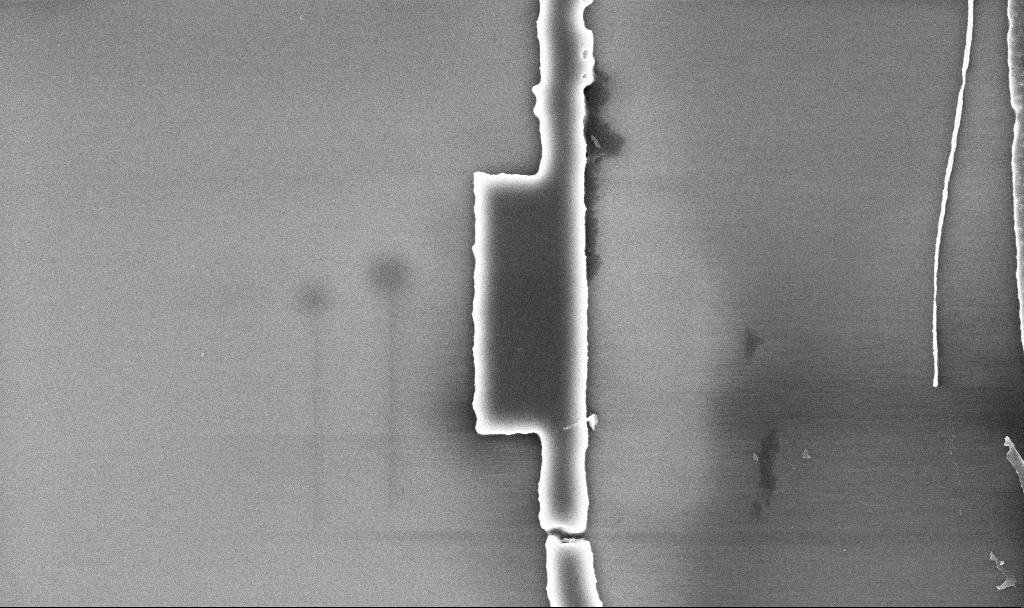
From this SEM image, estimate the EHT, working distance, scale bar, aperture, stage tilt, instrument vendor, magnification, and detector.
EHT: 5 kV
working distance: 5.2 mm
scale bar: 1000 nm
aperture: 30 µm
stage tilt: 0°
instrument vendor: Zeiss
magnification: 32.32 K X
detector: InLens